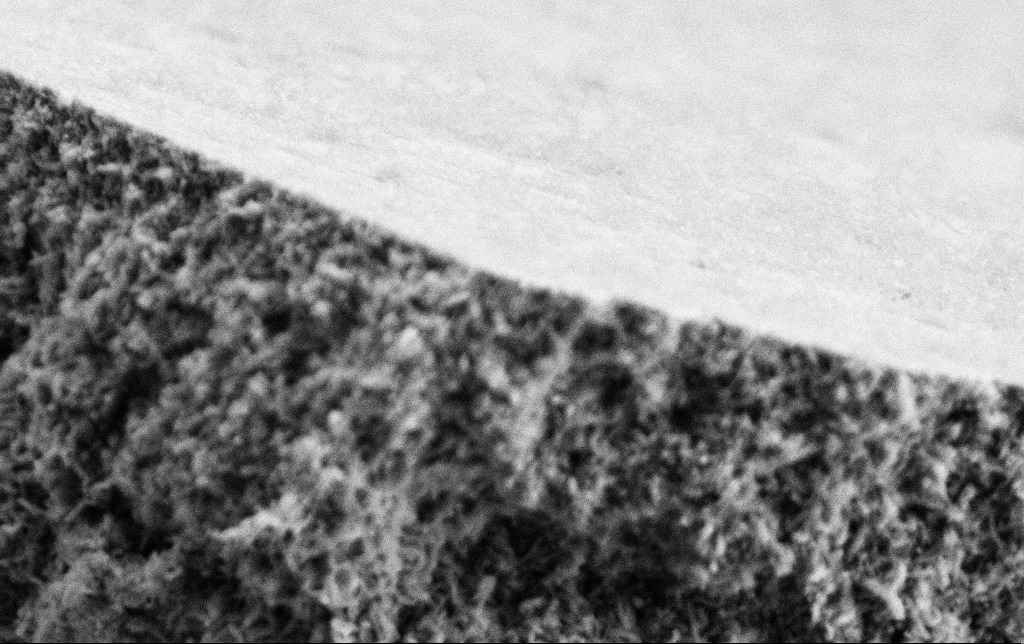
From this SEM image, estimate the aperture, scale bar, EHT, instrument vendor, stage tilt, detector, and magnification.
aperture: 30 µm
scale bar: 1000 nm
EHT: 2 kV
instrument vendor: Zeiss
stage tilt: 0°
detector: SE2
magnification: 50 K X